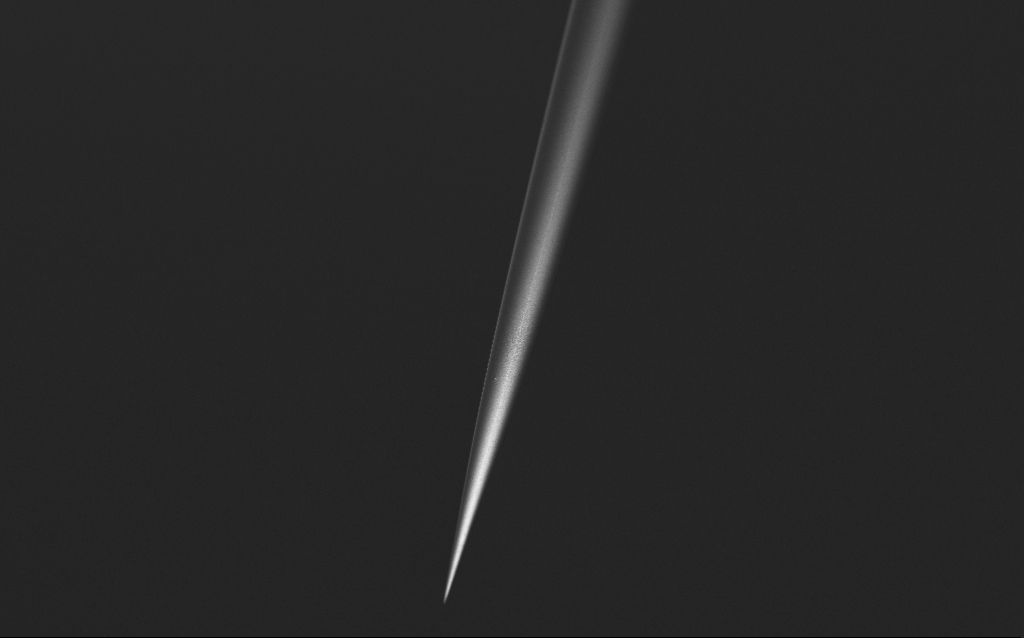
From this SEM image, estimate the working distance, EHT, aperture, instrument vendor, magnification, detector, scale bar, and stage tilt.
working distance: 5 mm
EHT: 2.5 kV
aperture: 30 µm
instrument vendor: Zeiss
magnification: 1 K X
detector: InLens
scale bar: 20000 nm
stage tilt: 45°